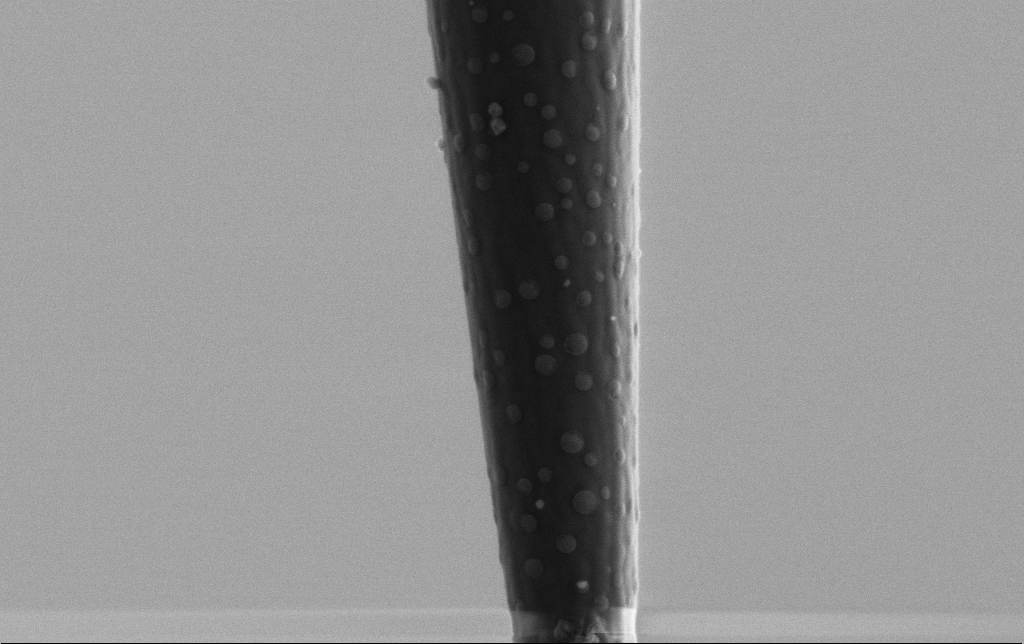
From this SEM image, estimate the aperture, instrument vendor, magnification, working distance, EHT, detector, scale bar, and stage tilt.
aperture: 30 µm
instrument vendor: Zeiss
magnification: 50 K X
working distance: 6.5 mm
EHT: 2 kV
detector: SE2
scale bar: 1000 nm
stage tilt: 0°